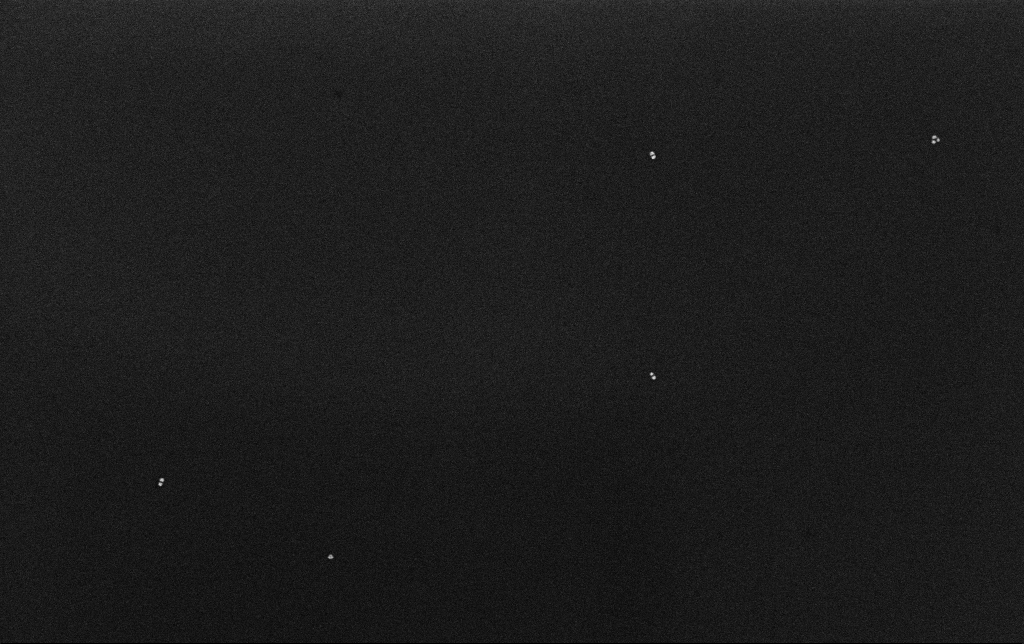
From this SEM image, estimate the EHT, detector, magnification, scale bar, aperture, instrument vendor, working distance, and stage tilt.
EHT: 10 kV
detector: InLens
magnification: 100 K X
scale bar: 200 nm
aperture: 30 µm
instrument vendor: Zeiss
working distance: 3.2 mm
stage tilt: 0°